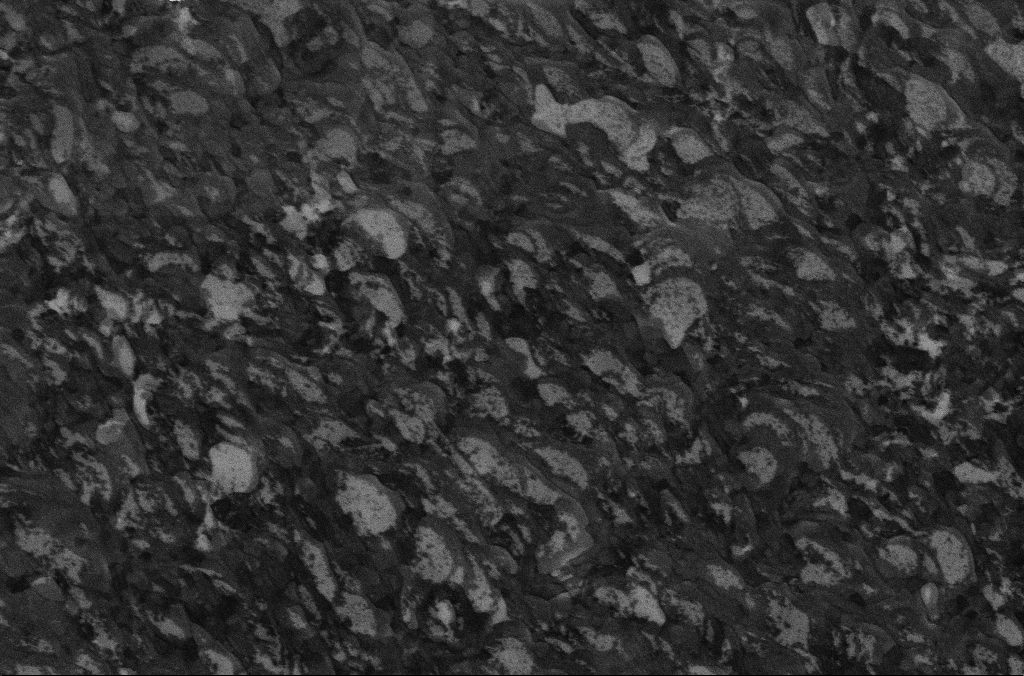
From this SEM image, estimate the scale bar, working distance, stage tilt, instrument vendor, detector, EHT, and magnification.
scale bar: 1000 nm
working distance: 6.7 mm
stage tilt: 0°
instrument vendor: Zeiss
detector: InLens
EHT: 5 kV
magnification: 20 K X